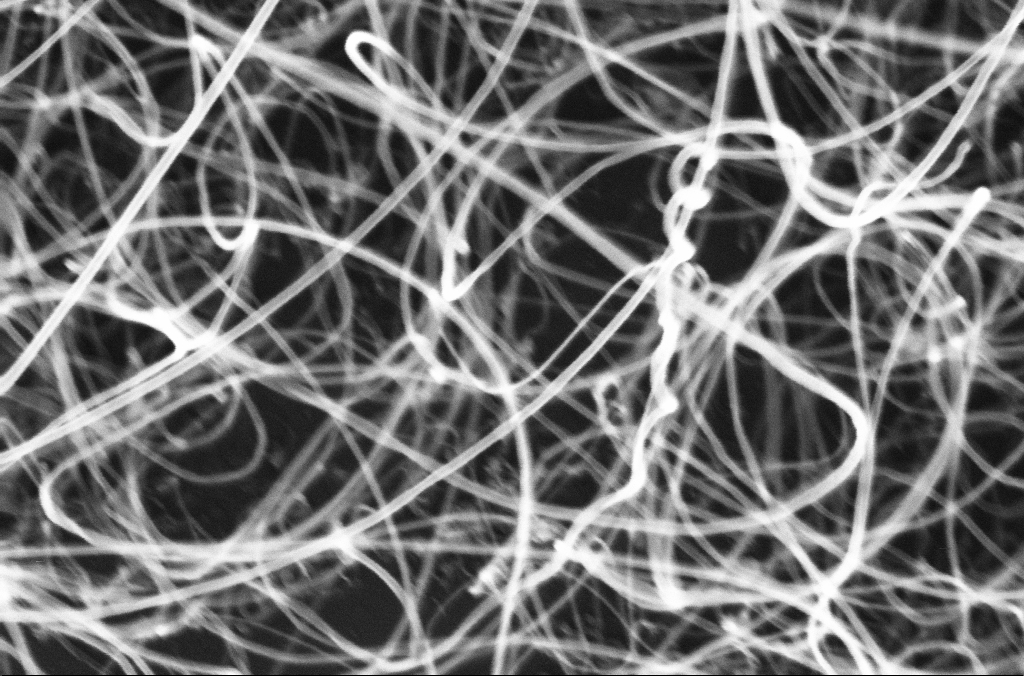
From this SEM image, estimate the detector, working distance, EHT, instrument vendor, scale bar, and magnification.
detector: InLens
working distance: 3.3 mm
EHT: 10 kV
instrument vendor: Zeiss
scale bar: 200 nm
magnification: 200 K X